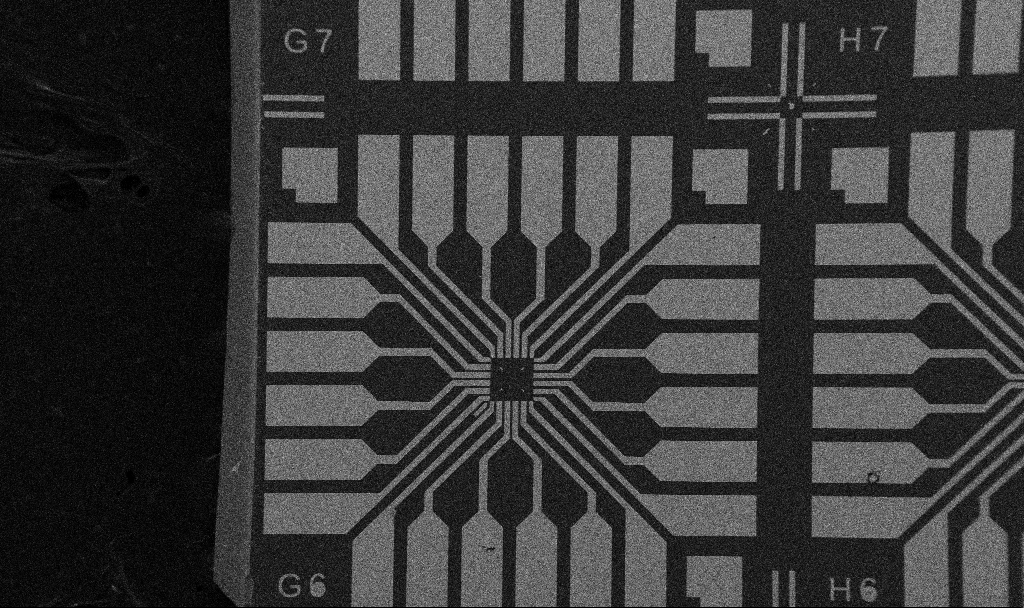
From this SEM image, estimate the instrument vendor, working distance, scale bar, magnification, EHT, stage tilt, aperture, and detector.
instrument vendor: Zeiss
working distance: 10.7 mm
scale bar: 200000 nm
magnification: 0.1 K X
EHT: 5 kV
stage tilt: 0°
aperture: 30 µm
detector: SE2